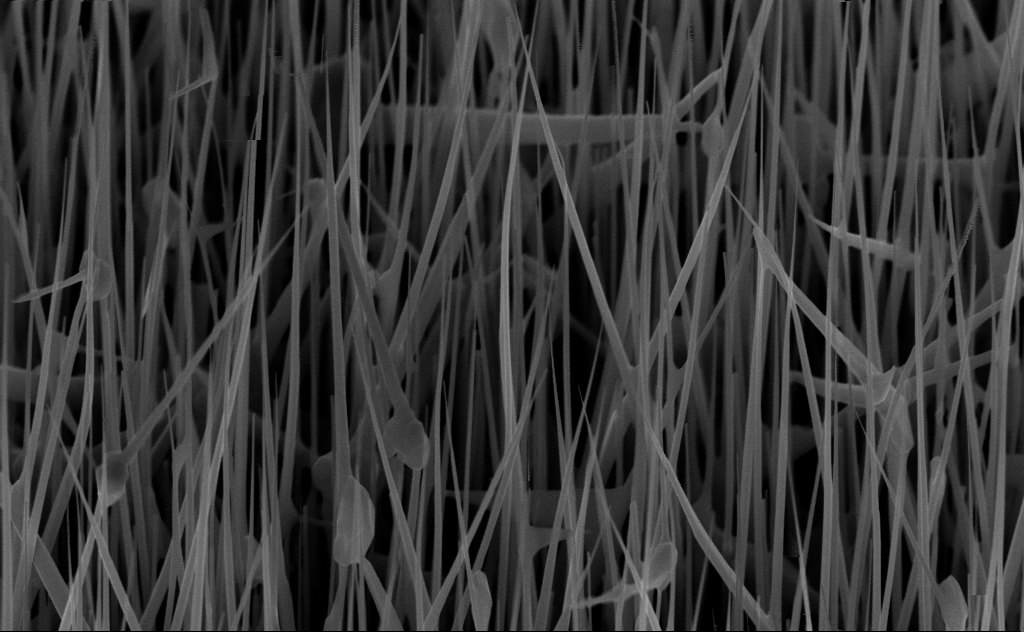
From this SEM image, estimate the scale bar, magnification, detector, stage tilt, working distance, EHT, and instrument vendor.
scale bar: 1000 nm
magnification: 40 K X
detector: InLens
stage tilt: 45°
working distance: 6 mm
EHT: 10 kV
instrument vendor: Zeiss